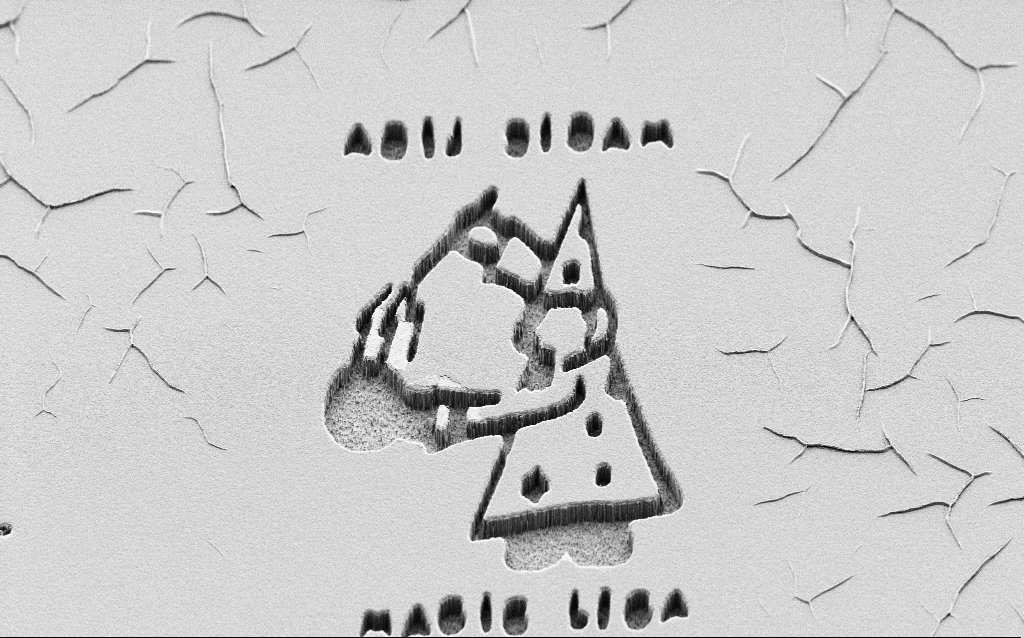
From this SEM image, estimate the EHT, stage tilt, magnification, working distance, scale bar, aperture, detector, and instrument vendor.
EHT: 2 kV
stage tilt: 45°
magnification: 1.35 K X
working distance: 8 mm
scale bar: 10000 nm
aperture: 30 µm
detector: SE2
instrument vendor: Zeiss